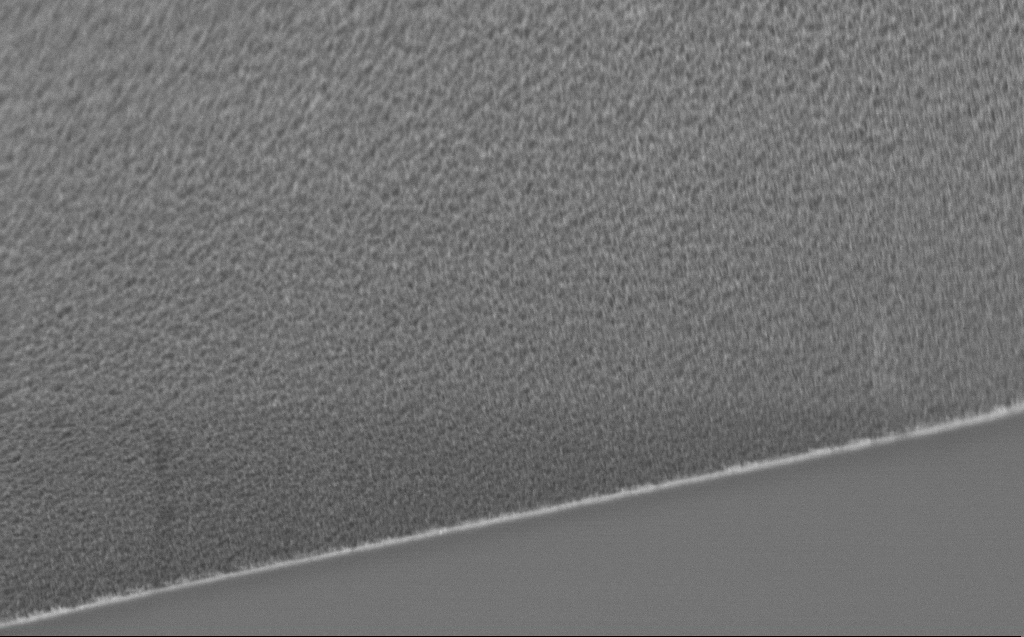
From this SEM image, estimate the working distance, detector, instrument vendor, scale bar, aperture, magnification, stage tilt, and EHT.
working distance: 4 mm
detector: InLens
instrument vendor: Zeiss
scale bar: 2000 nm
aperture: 20 µm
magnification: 34.09 K X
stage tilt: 44.9°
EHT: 1 kV